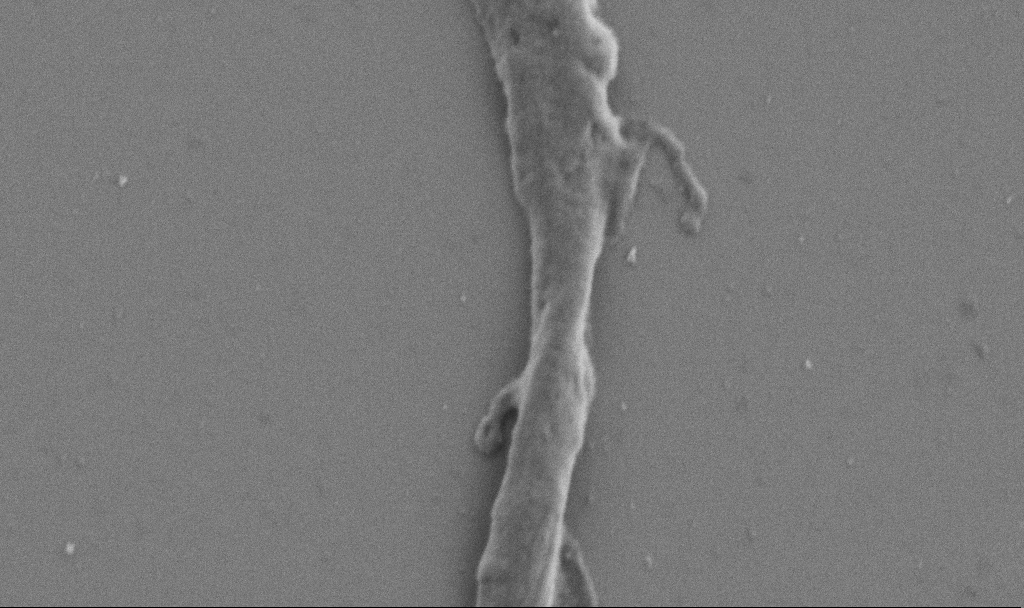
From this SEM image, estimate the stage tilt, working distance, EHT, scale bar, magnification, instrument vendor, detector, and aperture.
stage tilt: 0°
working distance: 6.9 mm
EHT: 1 kV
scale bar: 1000 nm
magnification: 40 K X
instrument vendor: Zeiss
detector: SE2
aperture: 30 µm